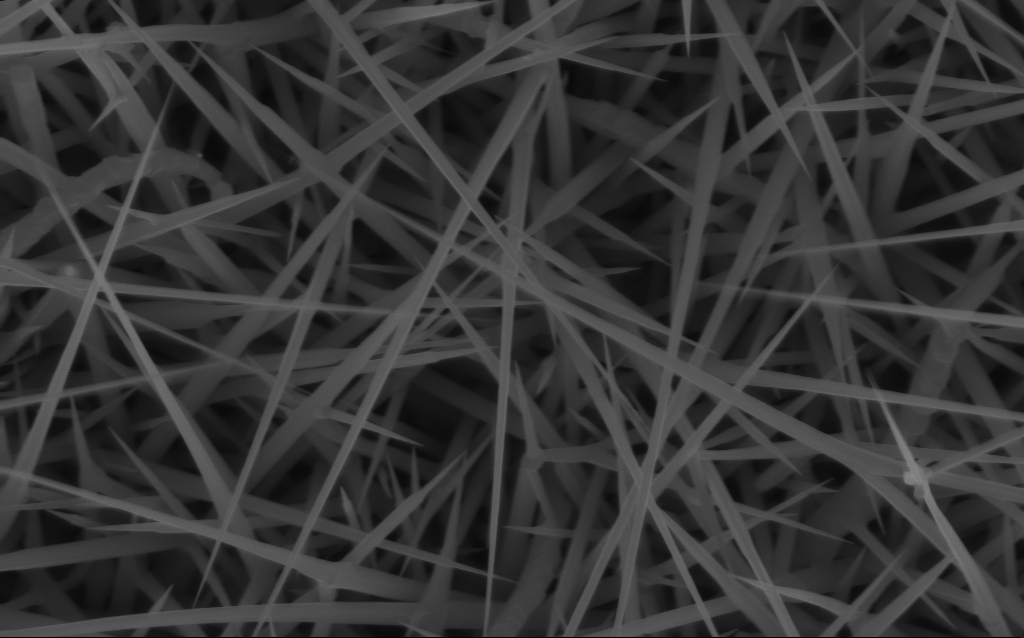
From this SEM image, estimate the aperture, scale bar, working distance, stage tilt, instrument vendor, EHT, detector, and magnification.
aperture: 30 µm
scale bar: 1000 nm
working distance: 7 mm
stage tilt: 0°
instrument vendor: Zeiss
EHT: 10 kV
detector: InLens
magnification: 40 K X